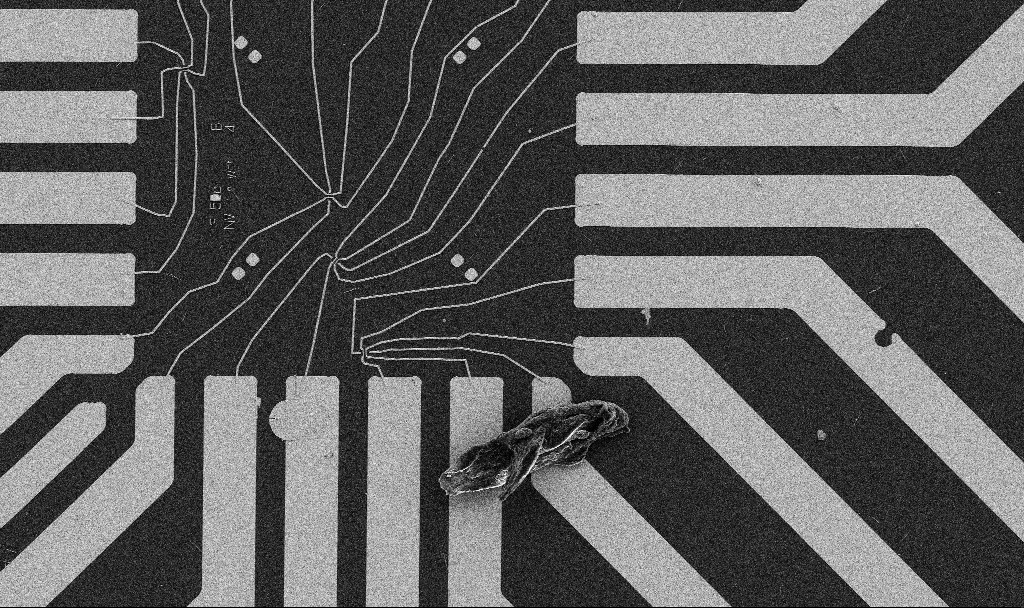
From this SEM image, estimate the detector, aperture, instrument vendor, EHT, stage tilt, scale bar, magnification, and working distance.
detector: SE2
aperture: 30 µm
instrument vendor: Zeiss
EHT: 5 kV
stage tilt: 0°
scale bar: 20000 nm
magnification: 1 K X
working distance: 10.7 mm